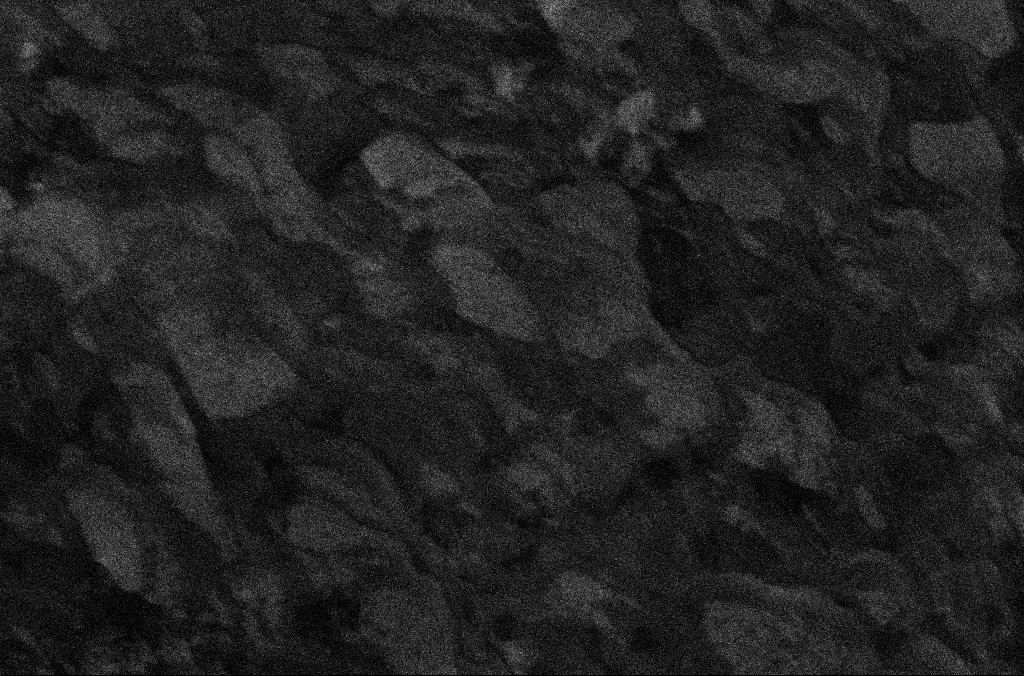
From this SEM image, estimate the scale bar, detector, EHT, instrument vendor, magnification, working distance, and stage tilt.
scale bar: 1000 nm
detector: InLens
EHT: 5 kV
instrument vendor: Zeiss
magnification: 50 K X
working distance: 6.7 mm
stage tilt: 0°